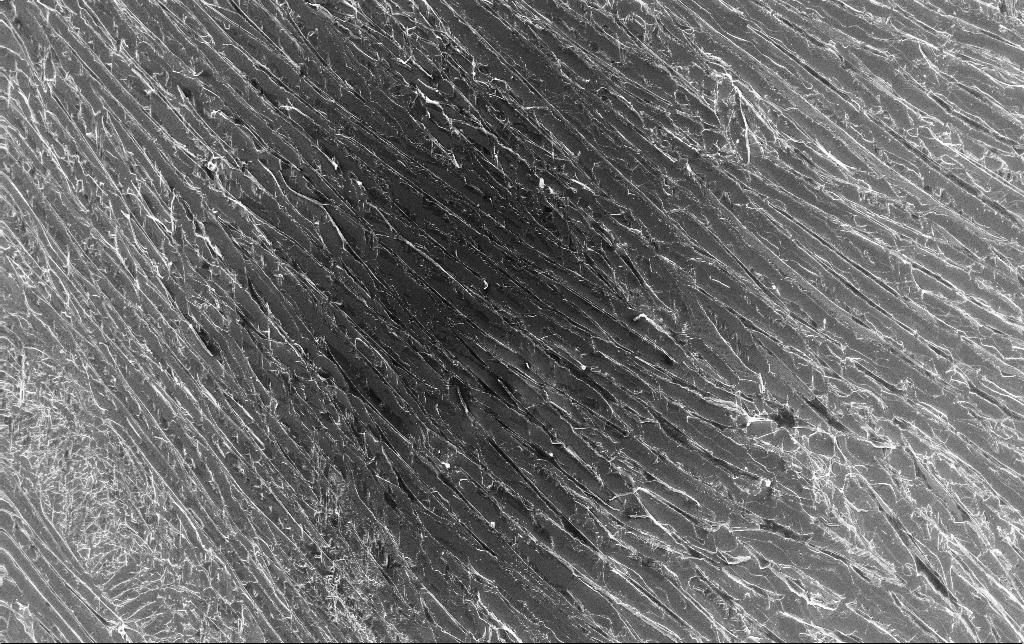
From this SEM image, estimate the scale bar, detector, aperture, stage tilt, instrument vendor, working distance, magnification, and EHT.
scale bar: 100000 nm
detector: InLens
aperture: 30 µm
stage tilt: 0°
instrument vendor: Zeiss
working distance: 3.2 mm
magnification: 0.341 K X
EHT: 10 kV